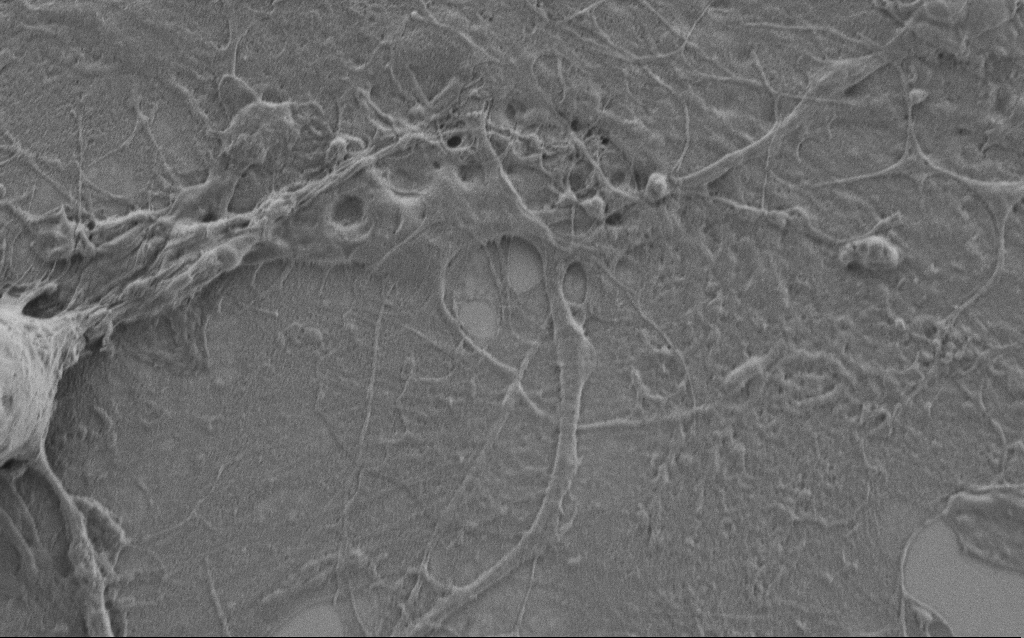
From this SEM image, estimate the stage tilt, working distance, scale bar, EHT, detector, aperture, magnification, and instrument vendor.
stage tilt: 0°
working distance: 5.9 mm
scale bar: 2000 nm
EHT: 1 kV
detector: SE2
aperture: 30 µm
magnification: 10 K X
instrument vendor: Zeiss